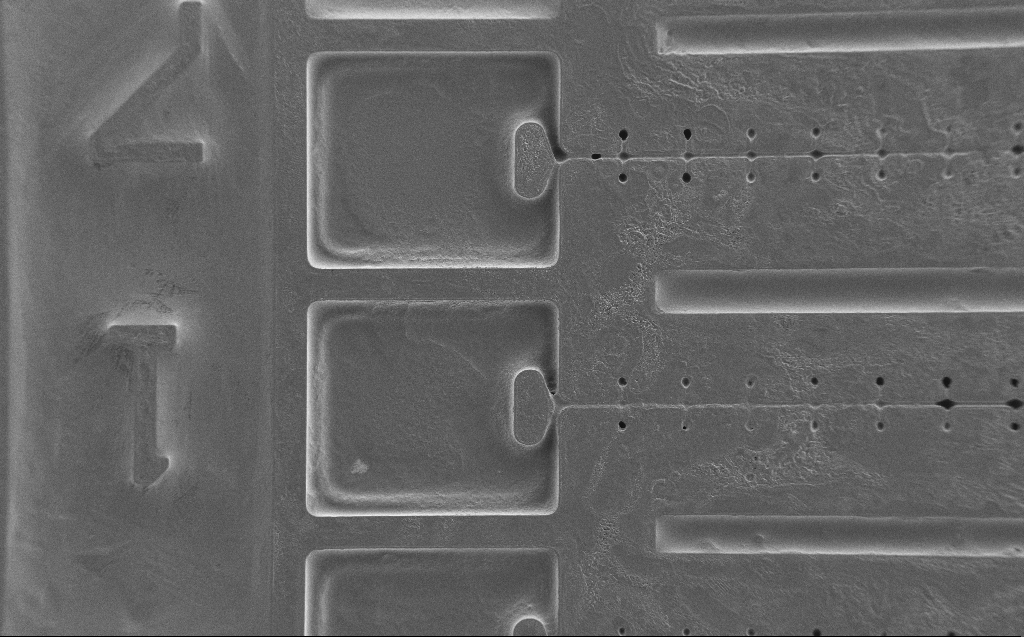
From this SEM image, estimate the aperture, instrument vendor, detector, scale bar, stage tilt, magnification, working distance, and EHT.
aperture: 30 µm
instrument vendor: Zeiss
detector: SE2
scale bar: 100000 nm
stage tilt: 0°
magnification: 0.234 K X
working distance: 7 mm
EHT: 2 kV